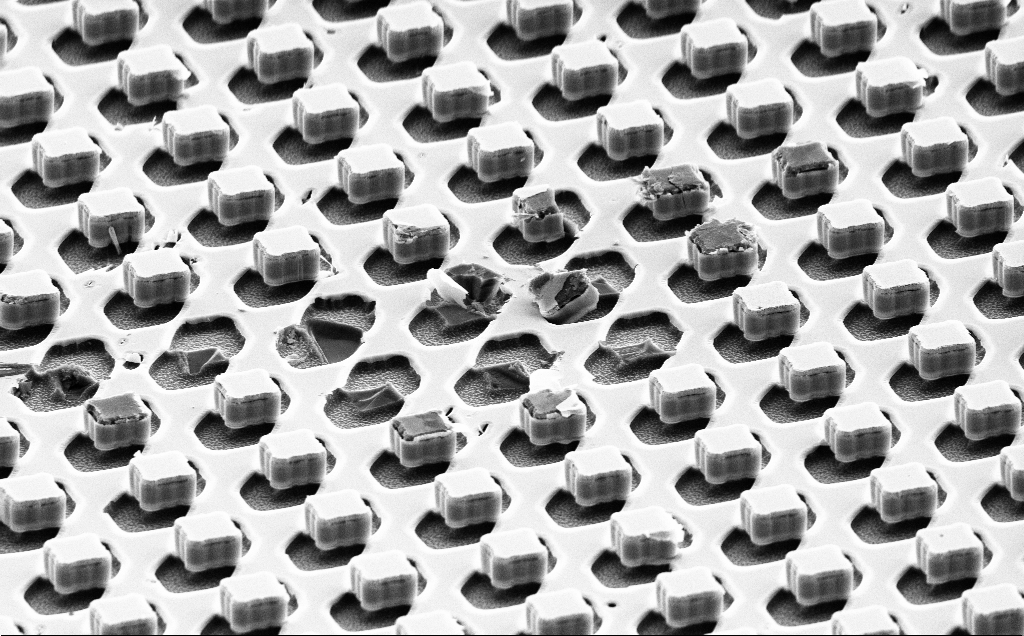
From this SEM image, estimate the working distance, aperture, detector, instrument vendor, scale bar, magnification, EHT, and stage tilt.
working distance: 12 mm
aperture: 30 µm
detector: SE2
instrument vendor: Zeiss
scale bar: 10000 nm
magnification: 2.52 K X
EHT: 10 kV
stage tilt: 61.7°